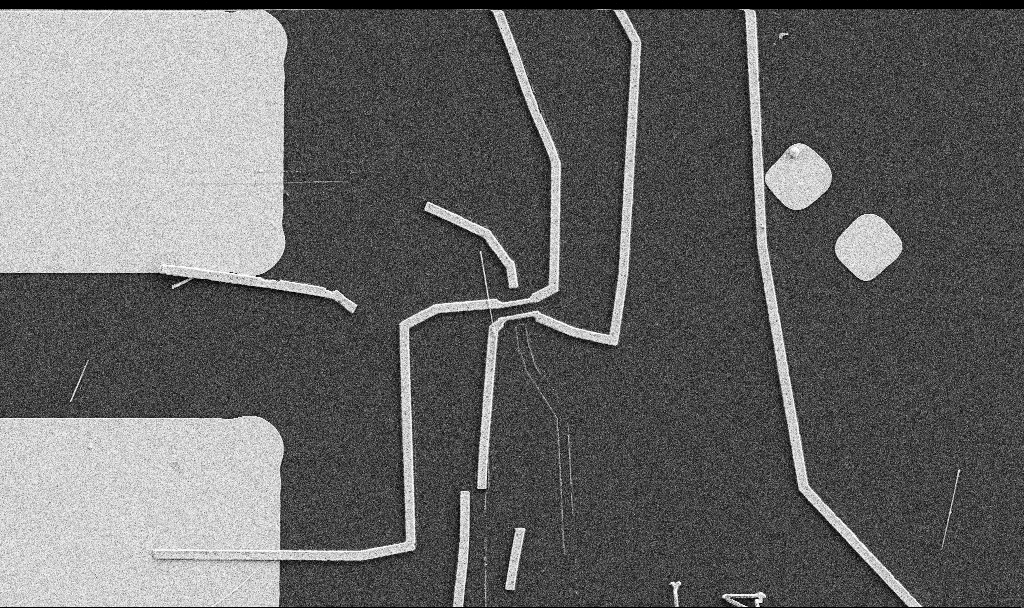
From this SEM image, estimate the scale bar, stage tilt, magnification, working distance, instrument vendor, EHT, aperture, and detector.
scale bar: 10000 nm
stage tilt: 0°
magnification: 5 K X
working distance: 10.7 mm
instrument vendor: Zeiss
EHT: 5 kV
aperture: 30 µm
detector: SE2